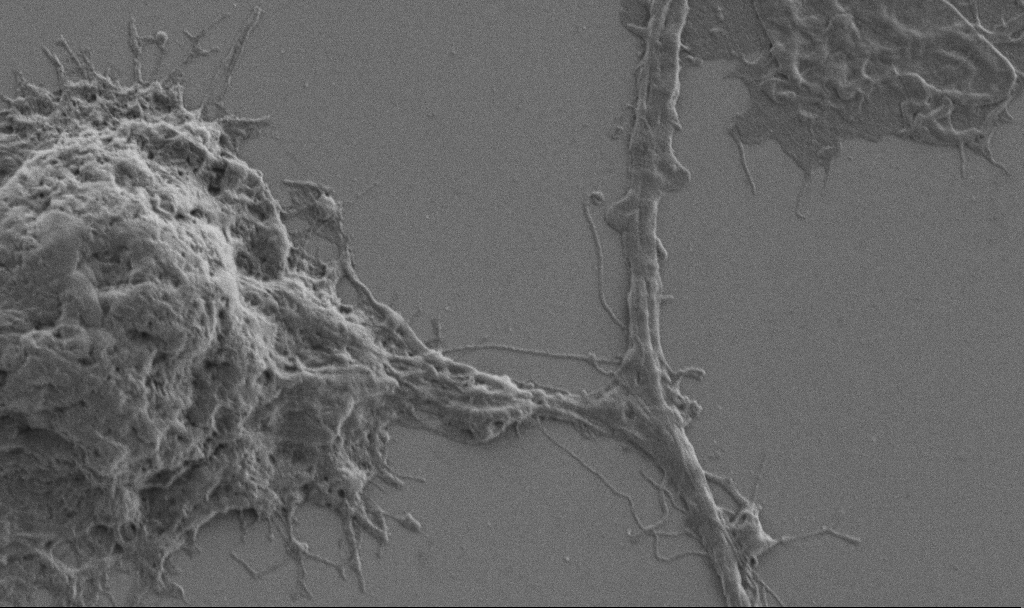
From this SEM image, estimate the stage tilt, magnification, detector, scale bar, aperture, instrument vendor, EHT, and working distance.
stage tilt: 0°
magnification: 10 K X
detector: SE2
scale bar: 2000 nm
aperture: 30 µm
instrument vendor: Zeiss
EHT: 1 kV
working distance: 6.9 mm